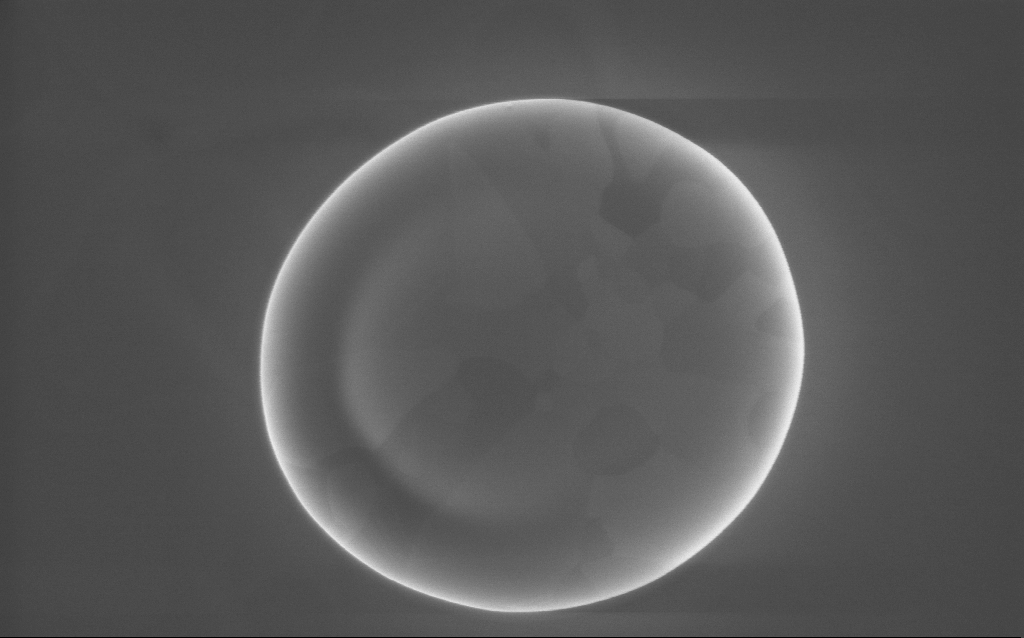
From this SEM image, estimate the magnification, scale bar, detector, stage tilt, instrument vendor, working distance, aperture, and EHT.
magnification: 58.76 K X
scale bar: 1000 nm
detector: InLens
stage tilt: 0°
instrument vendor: Zeiss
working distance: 2 mm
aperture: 30 µm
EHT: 10 kV